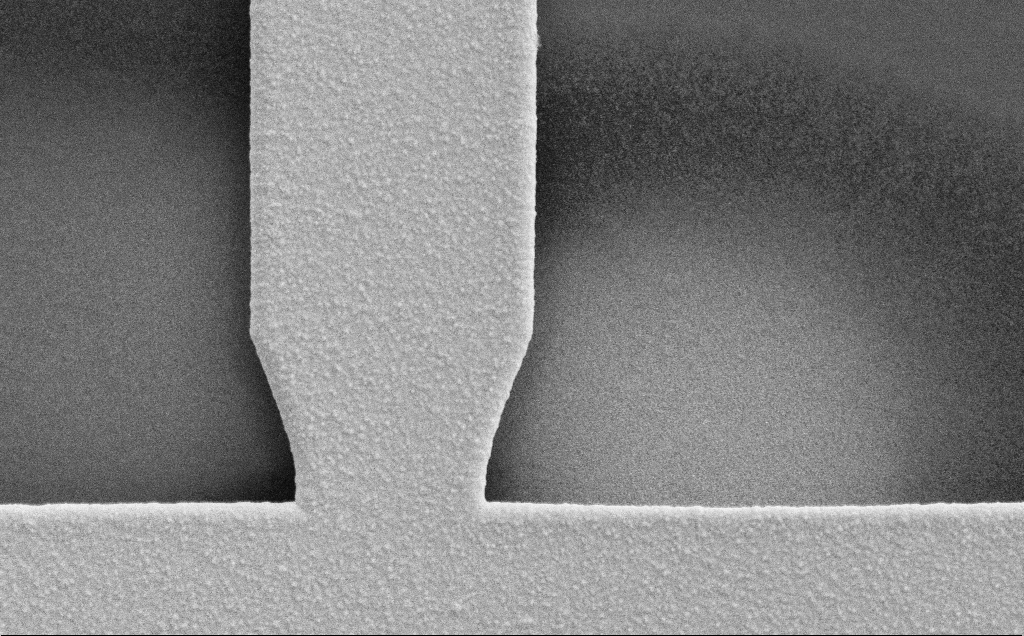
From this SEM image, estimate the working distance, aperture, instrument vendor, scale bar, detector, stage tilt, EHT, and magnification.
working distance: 12 mm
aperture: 30 µm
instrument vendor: Zeiss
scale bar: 2000 nm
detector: SE2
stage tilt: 0°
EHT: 10 kV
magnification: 9.08 K X